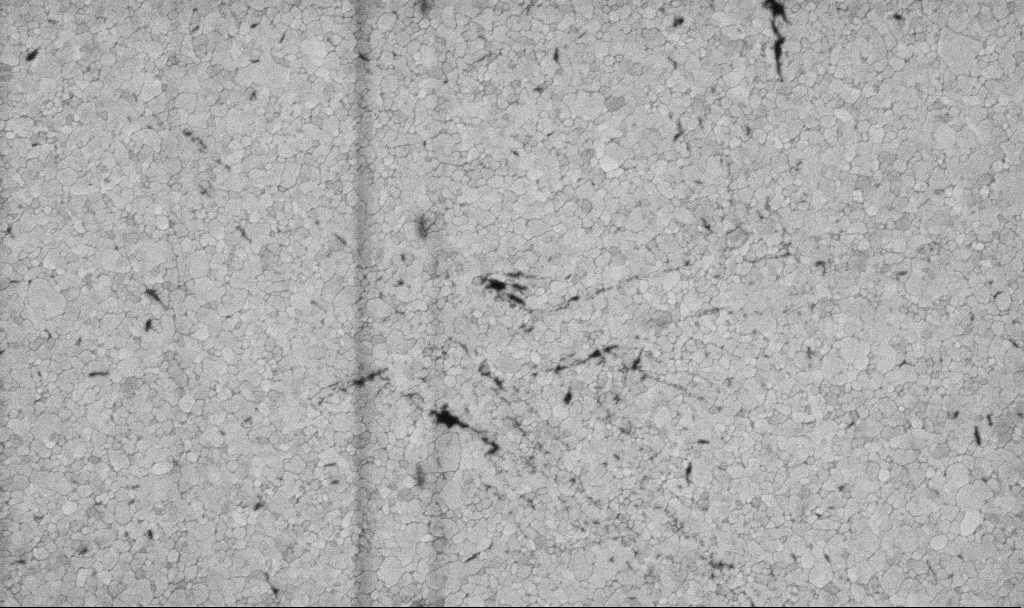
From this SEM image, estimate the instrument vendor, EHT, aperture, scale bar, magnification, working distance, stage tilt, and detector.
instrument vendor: Zeiss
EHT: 5 kV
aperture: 30 µm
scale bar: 1000 nm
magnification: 50.15 K X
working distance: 3.1 mm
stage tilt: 0°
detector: InLens